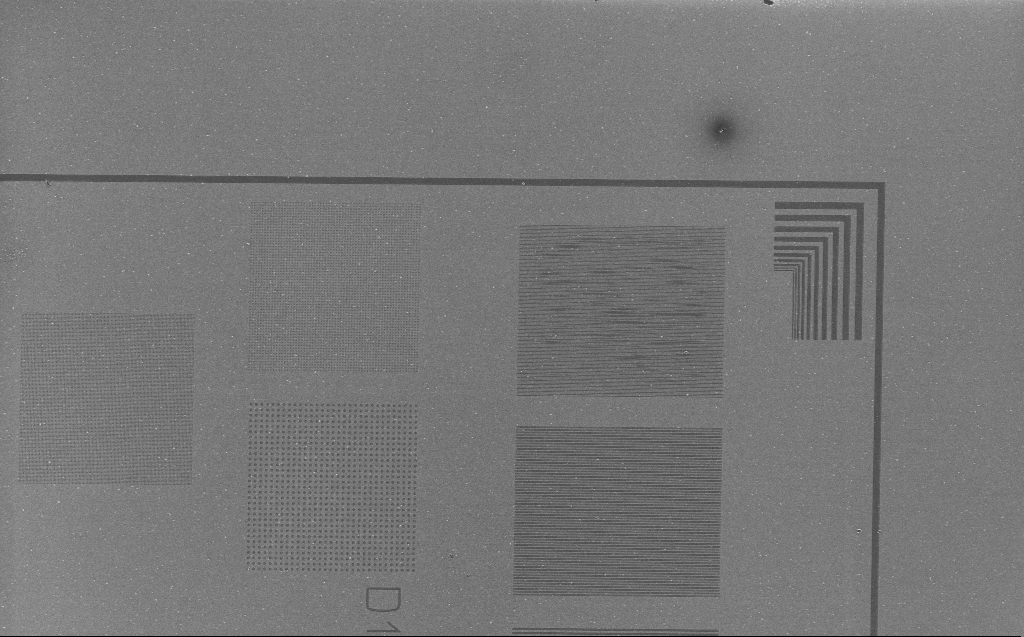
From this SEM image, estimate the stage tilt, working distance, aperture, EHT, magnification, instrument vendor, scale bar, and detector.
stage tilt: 0°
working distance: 4 mm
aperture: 30 µm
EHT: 1 kV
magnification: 2.55 K X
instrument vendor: Zeiss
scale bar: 10000 nm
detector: InLens